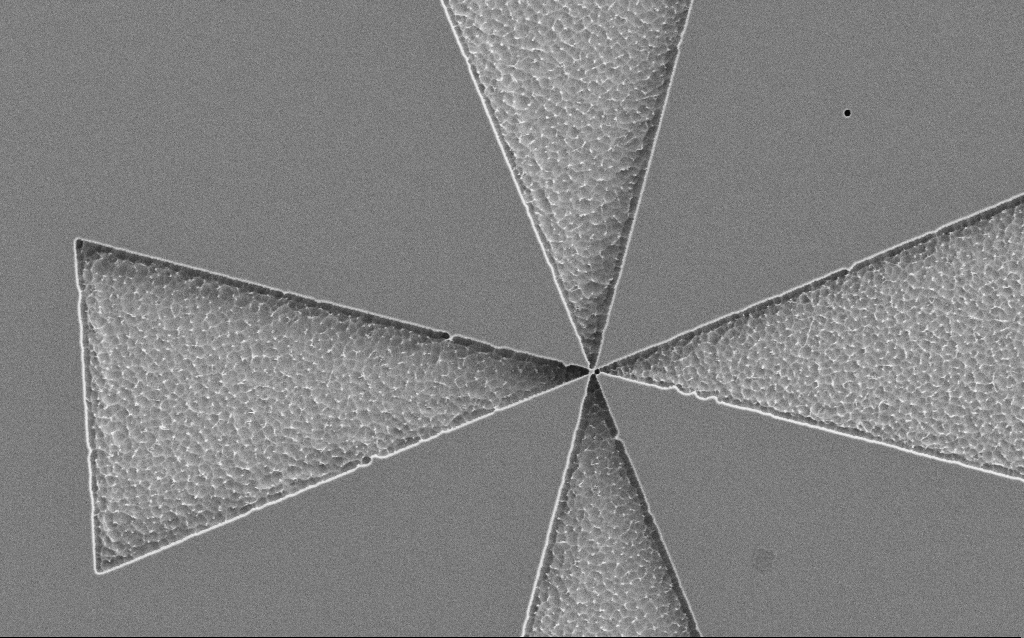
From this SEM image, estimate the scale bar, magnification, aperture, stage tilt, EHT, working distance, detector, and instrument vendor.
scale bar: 2000 nm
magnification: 12.43 K X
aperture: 30 µm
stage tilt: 0°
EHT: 2 kV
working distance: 5 mm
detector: SE2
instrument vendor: Zeiss